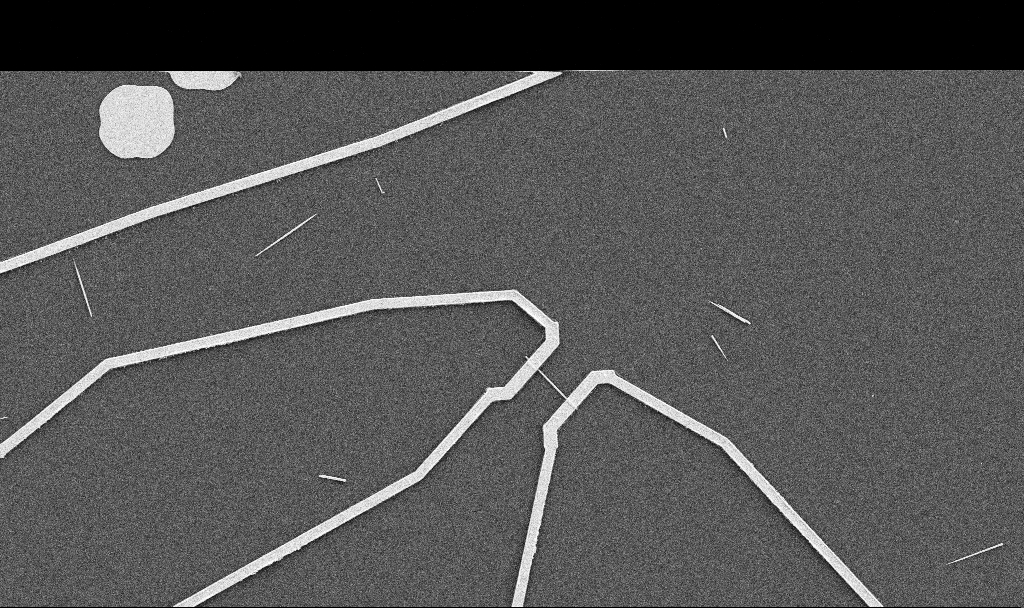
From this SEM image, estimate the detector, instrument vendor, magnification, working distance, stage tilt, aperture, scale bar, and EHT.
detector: SE2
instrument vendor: Zeiss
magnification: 5 K X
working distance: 10.7 mm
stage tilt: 0°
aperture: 30 µm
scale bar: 10000 nm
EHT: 5 kV